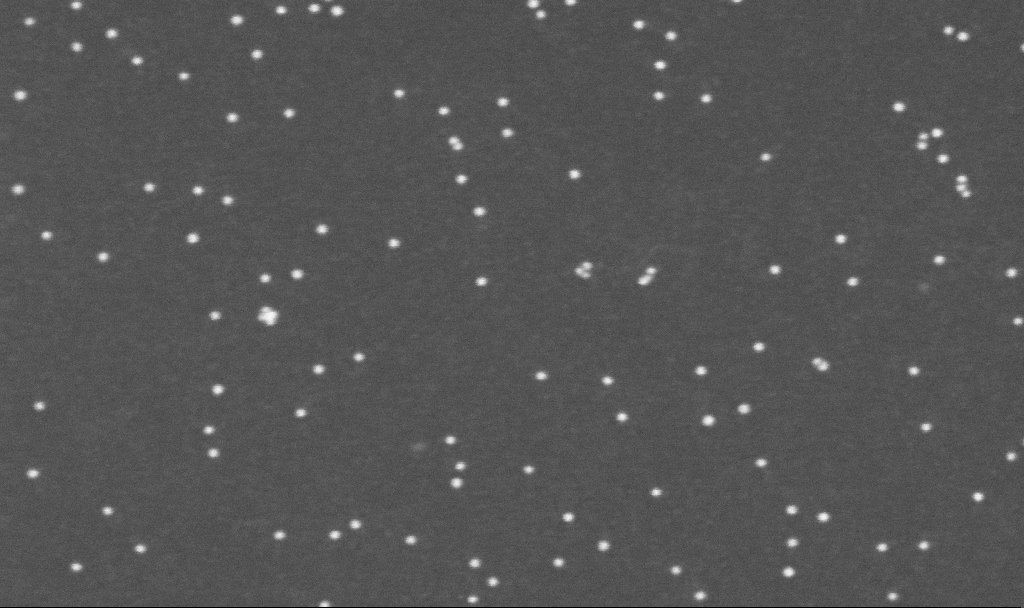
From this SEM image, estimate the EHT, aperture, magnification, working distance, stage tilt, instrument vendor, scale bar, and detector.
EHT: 10 kV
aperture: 30 µm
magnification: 300 K X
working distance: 3.3 mm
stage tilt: -0°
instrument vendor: Zeiss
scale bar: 200 nm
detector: InLens